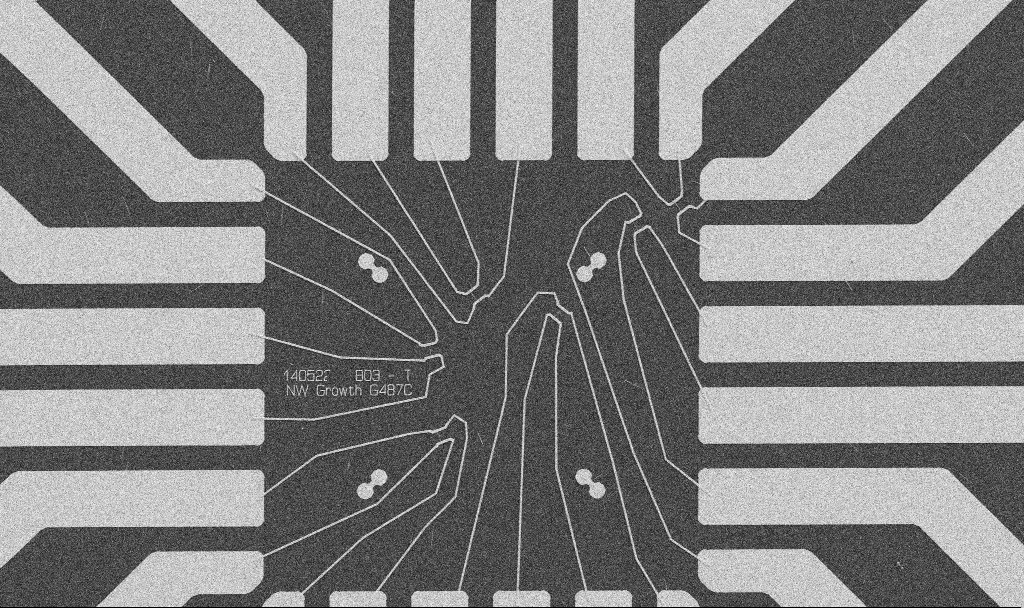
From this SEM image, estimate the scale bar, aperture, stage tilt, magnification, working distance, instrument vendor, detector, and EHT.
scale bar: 20000 nm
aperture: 30 µm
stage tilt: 0°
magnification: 1 K X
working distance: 10.6 mm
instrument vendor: Zeiss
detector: SE2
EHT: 5 kV